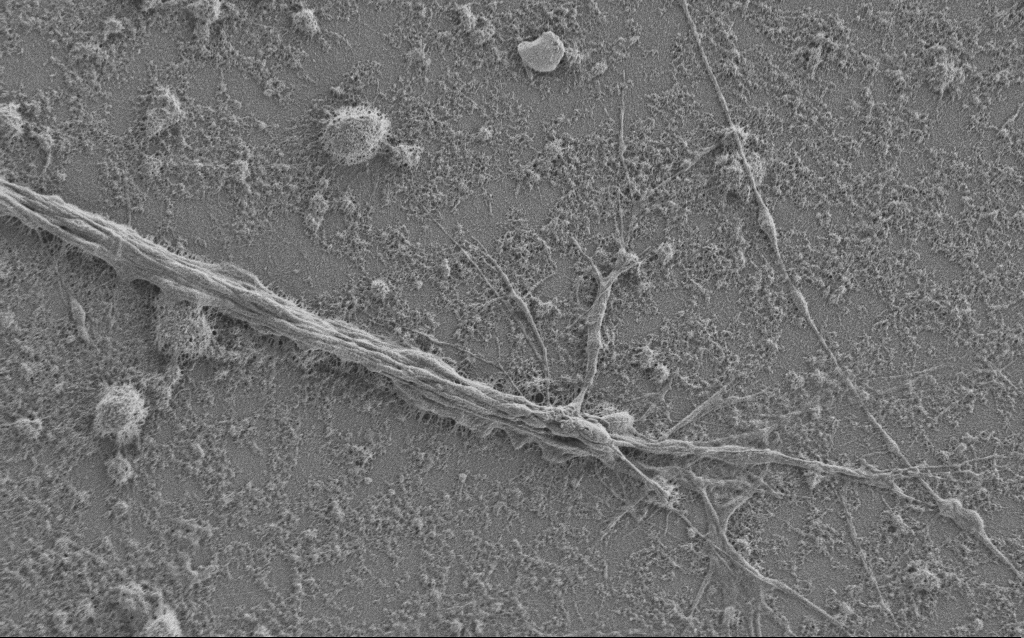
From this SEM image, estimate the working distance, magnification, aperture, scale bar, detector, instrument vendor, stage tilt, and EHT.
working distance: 6 mm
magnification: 6 K X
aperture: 30 µm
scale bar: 10000 nm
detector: SE2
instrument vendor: Zeiss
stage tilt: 0°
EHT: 1 kV